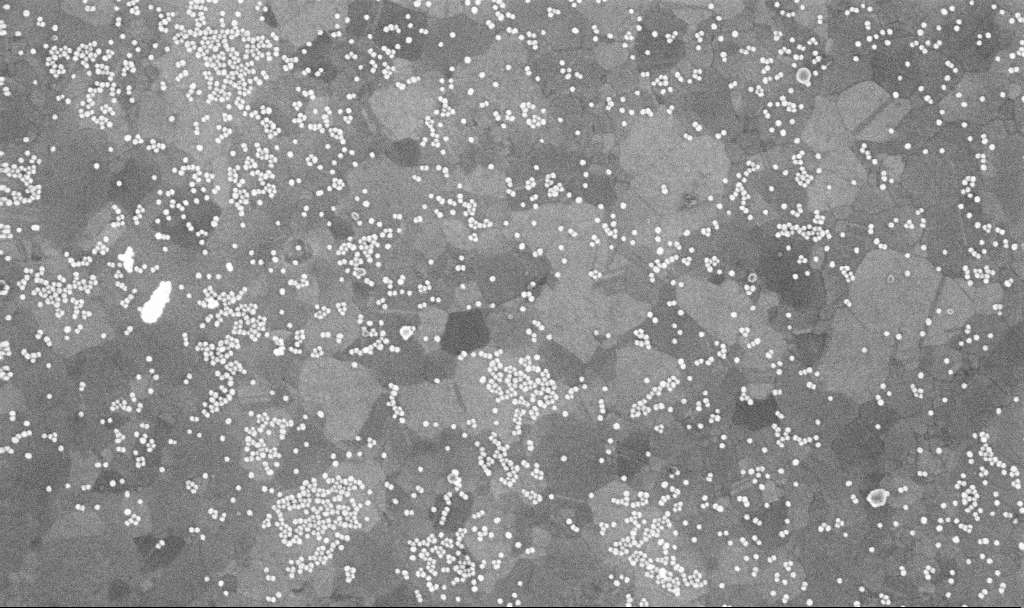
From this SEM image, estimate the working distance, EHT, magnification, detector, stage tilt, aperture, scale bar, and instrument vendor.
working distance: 3.7 mm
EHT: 10 kV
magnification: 100 K X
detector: InLens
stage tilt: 0°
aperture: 30 µm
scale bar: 200 nm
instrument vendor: Zeiss